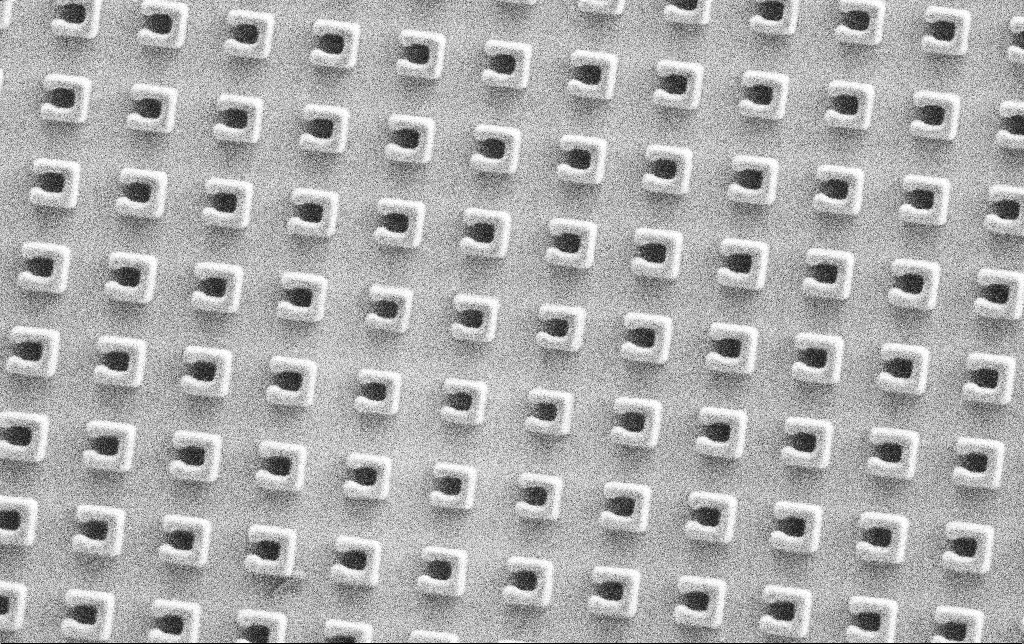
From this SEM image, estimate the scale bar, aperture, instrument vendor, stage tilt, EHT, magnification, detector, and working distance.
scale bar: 2000 nm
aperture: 30 µm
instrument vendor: Zeiss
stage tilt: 0°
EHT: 1.5 kV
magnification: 31.57 K X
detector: SE2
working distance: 7.4 mm